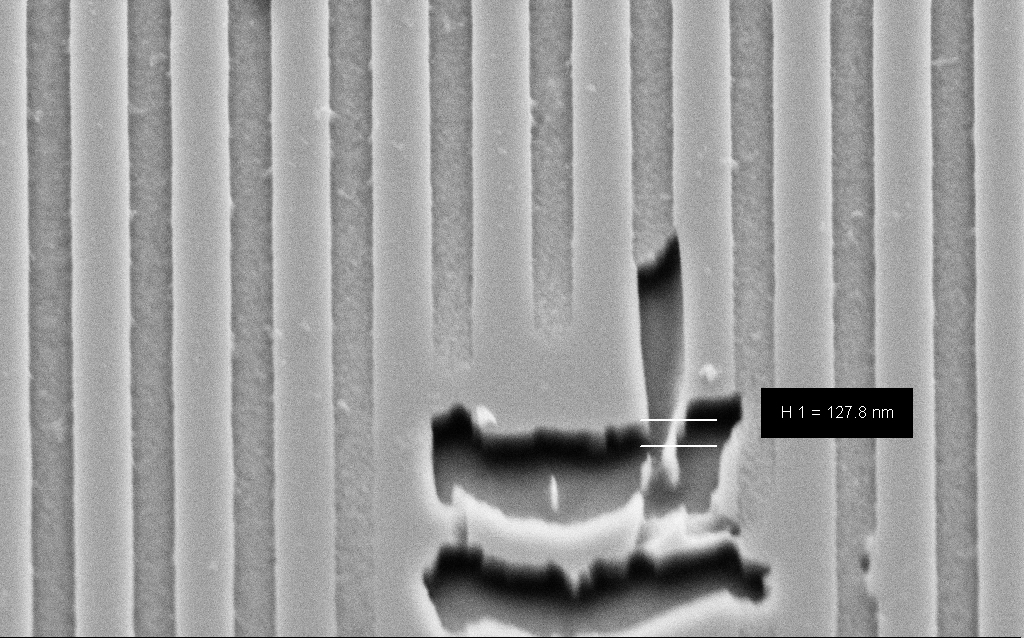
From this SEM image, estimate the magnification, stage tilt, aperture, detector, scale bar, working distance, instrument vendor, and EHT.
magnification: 74.73 K X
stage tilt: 45°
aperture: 30 µm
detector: SE2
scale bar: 200 nm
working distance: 6 mm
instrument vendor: Zeiss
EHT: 3 kV